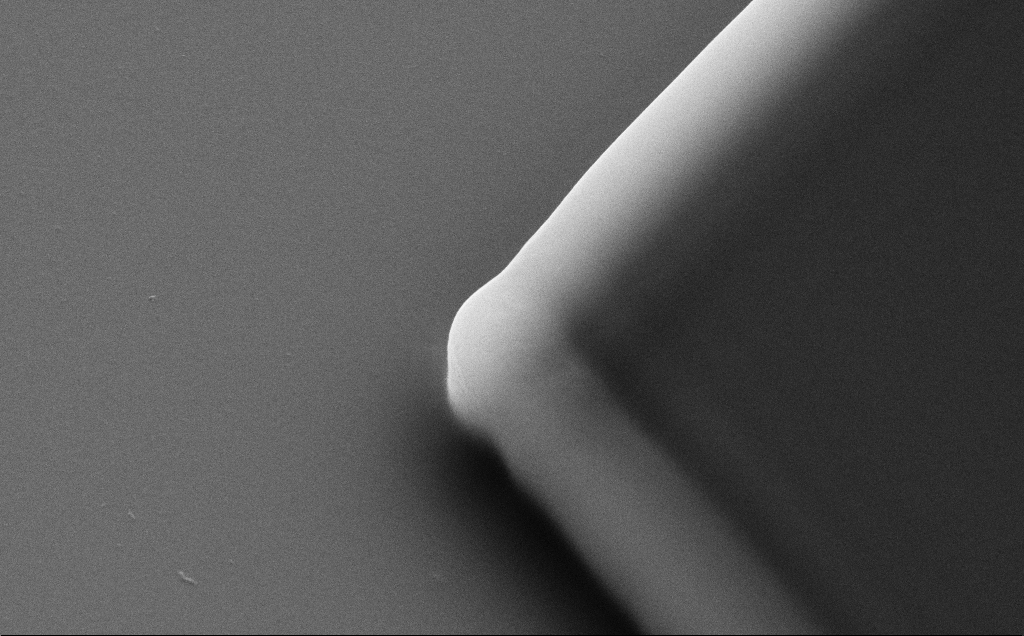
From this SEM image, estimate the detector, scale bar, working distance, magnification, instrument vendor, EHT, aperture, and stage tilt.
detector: SE2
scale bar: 1000 nm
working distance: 8 mm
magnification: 31.61 K X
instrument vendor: Zeiss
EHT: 10 kV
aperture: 30 µm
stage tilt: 35°